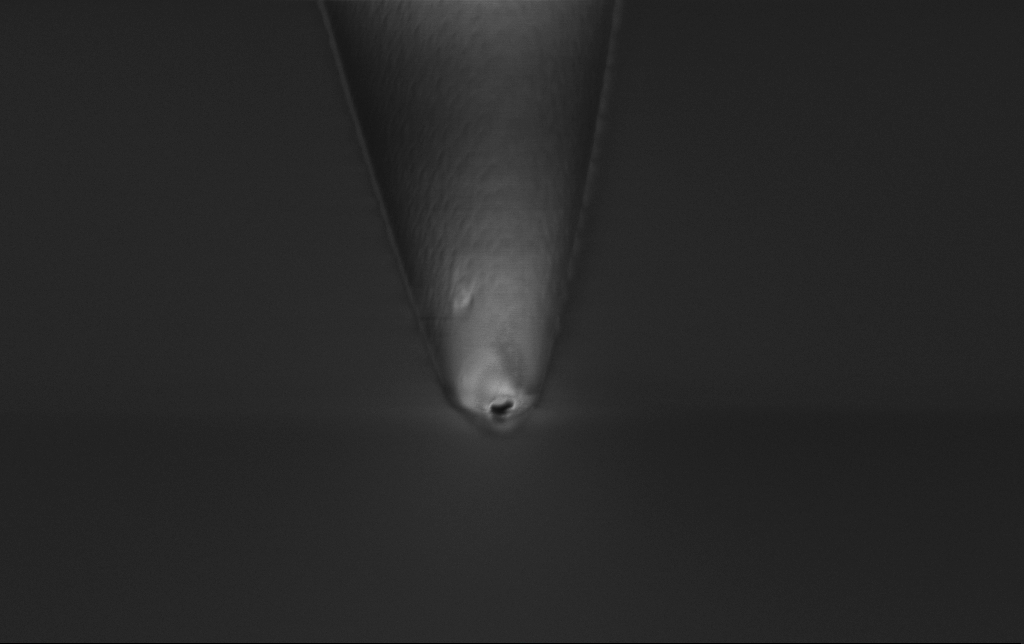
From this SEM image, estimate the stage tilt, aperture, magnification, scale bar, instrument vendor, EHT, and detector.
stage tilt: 45°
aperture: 30 µm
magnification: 25 K X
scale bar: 2000 nm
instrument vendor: Zeiss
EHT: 1 kV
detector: InLens